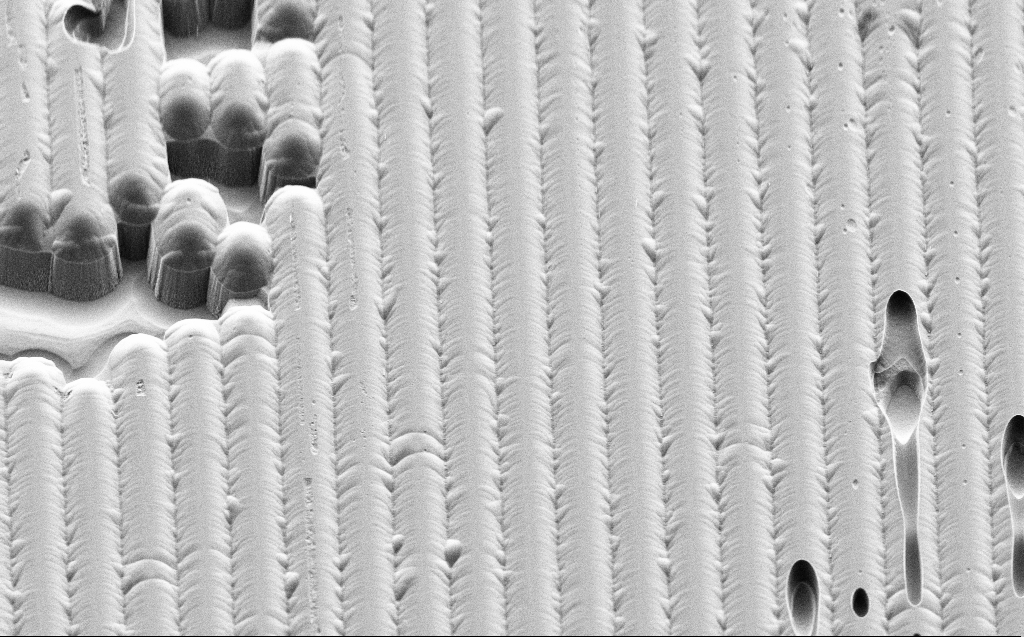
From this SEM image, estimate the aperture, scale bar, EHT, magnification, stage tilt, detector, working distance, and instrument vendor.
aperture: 30 µm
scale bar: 10000 nm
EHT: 3 kV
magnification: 2 K X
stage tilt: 45°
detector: SE2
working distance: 8 mm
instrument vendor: Zeiss